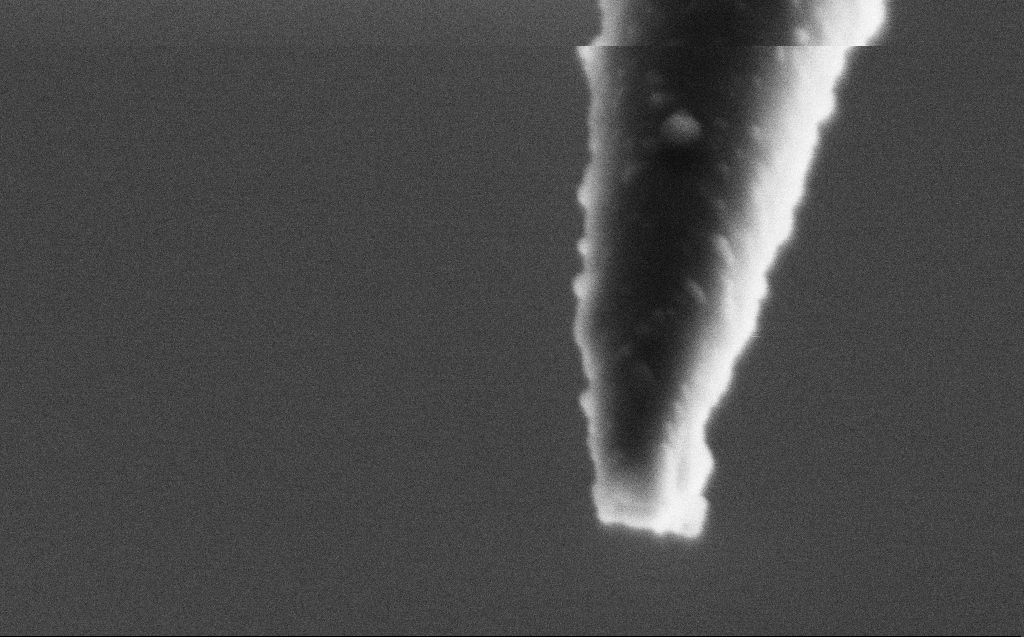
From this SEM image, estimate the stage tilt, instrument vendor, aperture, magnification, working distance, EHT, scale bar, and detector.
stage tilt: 45°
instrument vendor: Zeiss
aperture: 30 µm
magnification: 250 K X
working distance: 4 mm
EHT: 2 kV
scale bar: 200 nm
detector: SE2